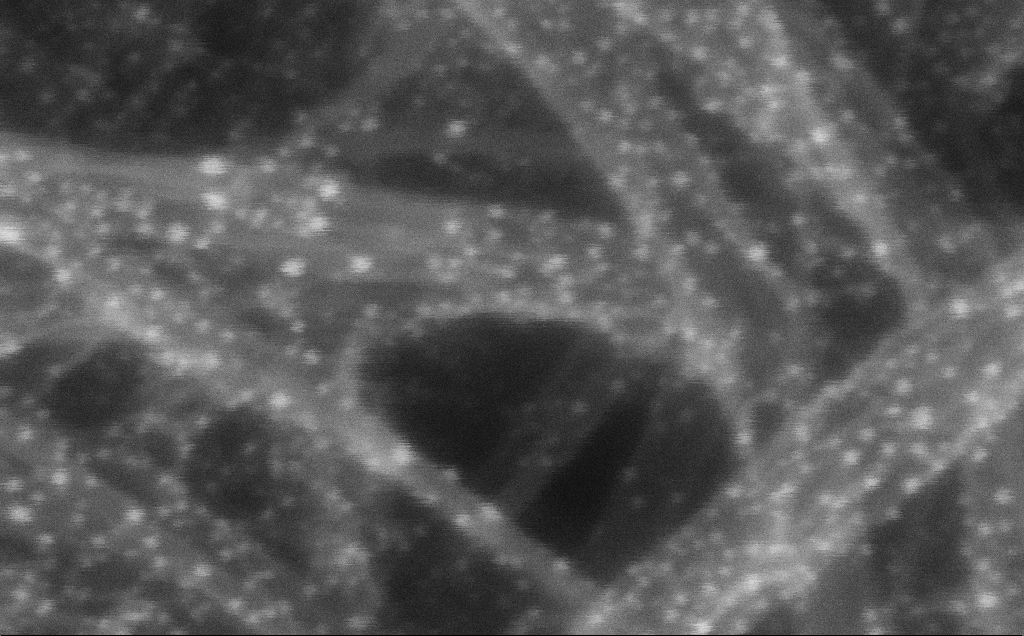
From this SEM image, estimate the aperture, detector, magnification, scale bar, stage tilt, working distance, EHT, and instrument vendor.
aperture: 30 µm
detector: InLens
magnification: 1031.08 K X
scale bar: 20 nm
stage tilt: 0°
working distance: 3 mm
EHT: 10 kV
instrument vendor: Zeiss